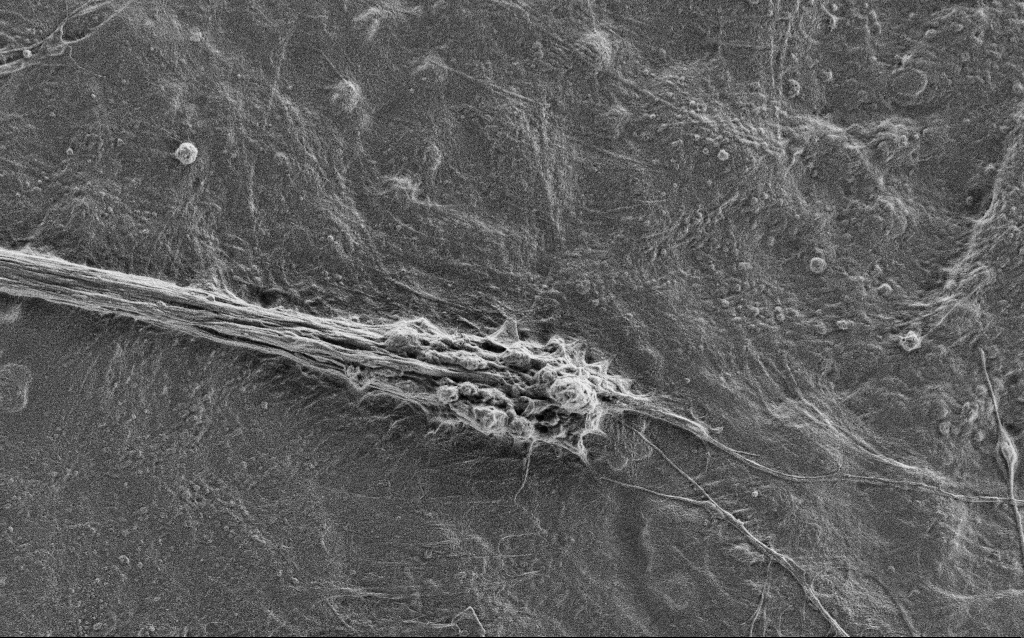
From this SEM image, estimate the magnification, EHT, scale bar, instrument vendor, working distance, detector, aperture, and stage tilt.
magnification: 5 K X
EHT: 0.9 kV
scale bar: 10000 nm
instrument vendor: Zeiss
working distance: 4 mm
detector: SE2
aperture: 30 µm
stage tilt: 0°